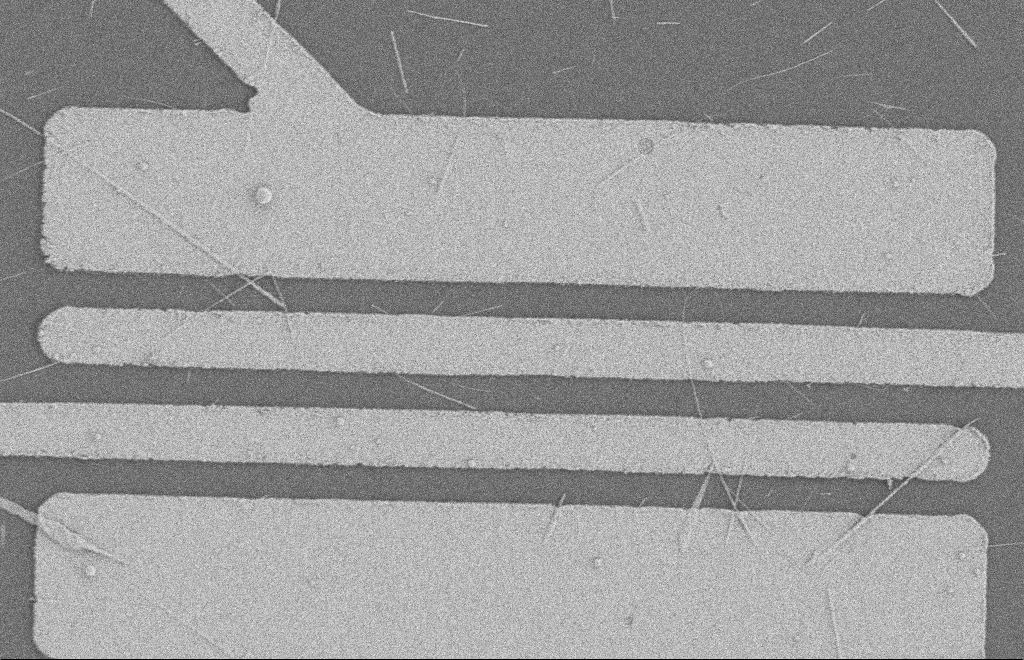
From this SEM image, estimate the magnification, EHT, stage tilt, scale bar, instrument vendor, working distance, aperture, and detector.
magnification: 5.71 K X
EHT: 2 kV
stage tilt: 0°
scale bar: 2000 nm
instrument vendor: Zeiss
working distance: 8 mm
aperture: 20 µm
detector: SE2